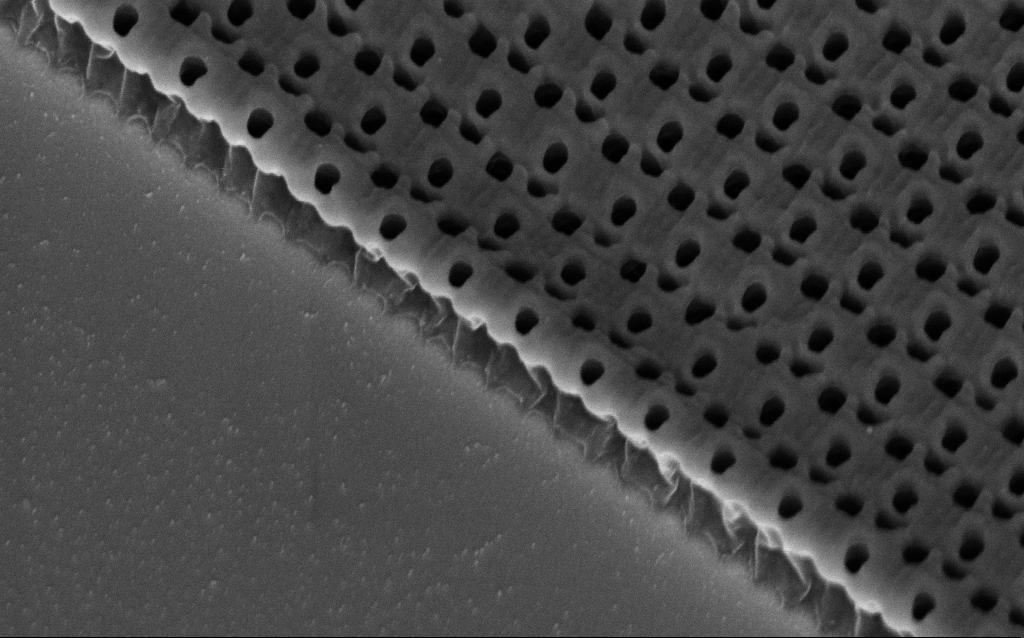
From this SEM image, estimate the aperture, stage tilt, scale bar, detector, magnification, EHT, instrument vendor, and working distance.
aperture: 30 µm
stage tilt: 45°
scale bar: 1000 nm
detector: InLens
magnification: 65.98 K X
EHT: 3 kV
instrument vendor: Zeiss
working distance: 7.7 mm